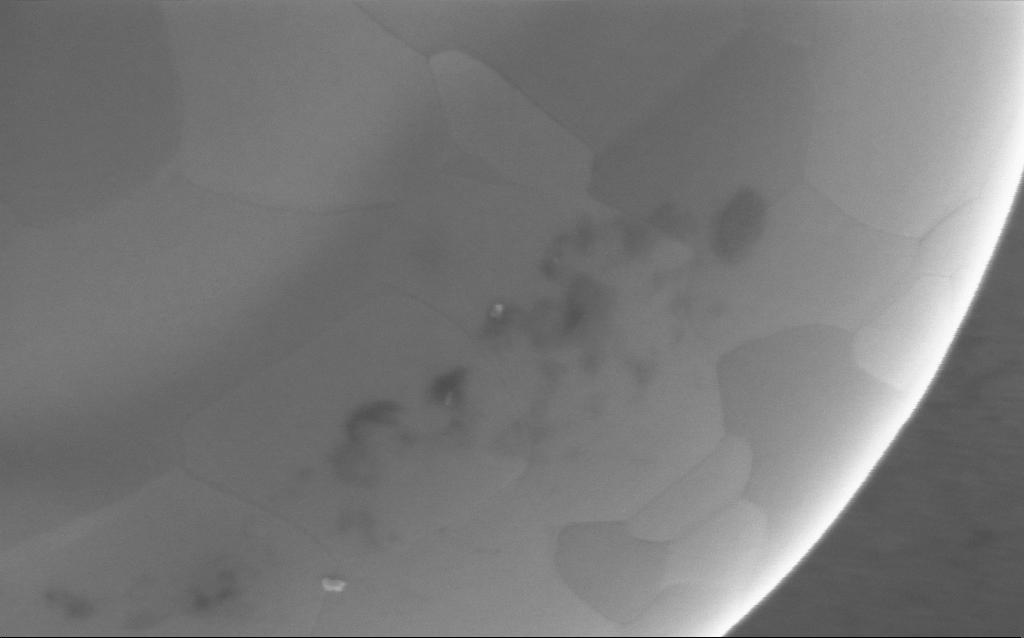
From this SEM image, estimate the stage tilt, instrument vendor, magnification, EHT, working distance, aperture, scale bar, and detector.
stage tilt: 0°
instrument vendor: Zeiss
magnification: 212.01 K X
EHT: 5 kV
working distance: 4 mm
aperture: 30 µm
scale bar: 200 nm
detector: InLens